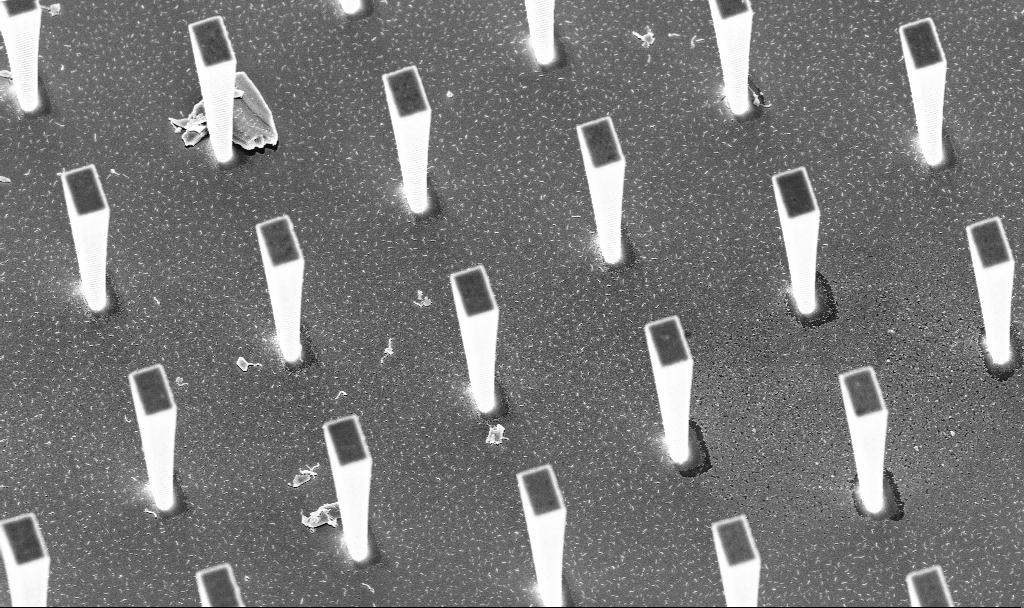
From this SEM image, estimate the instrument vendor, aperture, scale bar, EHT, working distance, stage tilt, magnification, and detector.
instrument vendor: Zeiss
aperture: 30 µm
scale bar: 10000 nm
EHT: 5 kV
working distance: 5.2 mm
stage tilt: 20°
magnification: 6.7 K X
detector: InLens